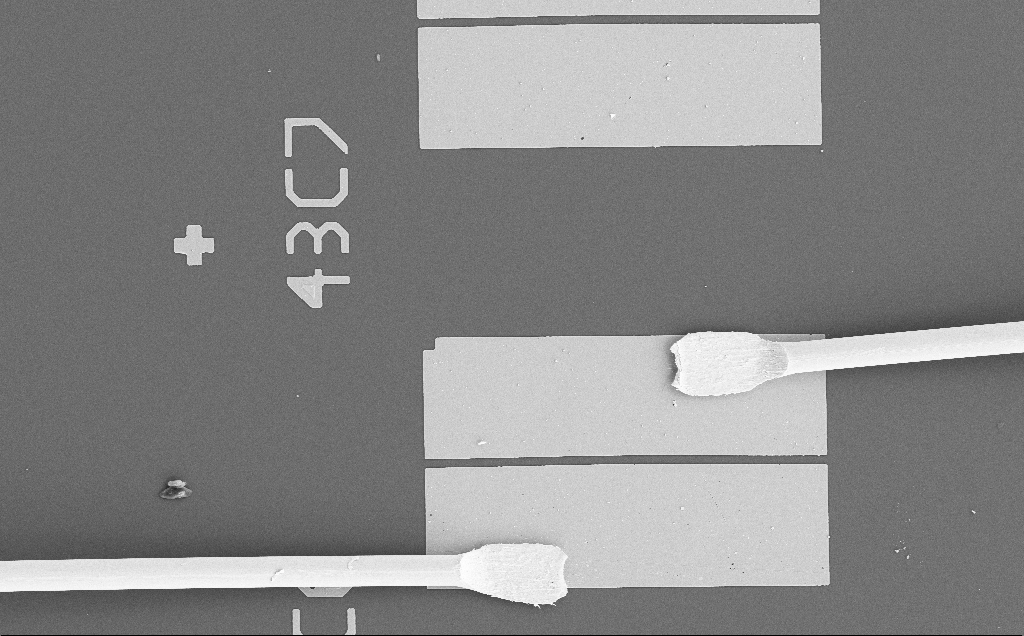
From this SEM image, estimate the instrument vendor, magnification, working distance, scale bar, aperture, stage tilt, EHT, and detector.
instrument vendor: Zeiss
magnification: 0.463 K X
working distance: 11 mm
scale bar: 100000 nm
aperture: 30 µm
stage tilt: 0°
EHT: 15 kV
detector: SE2